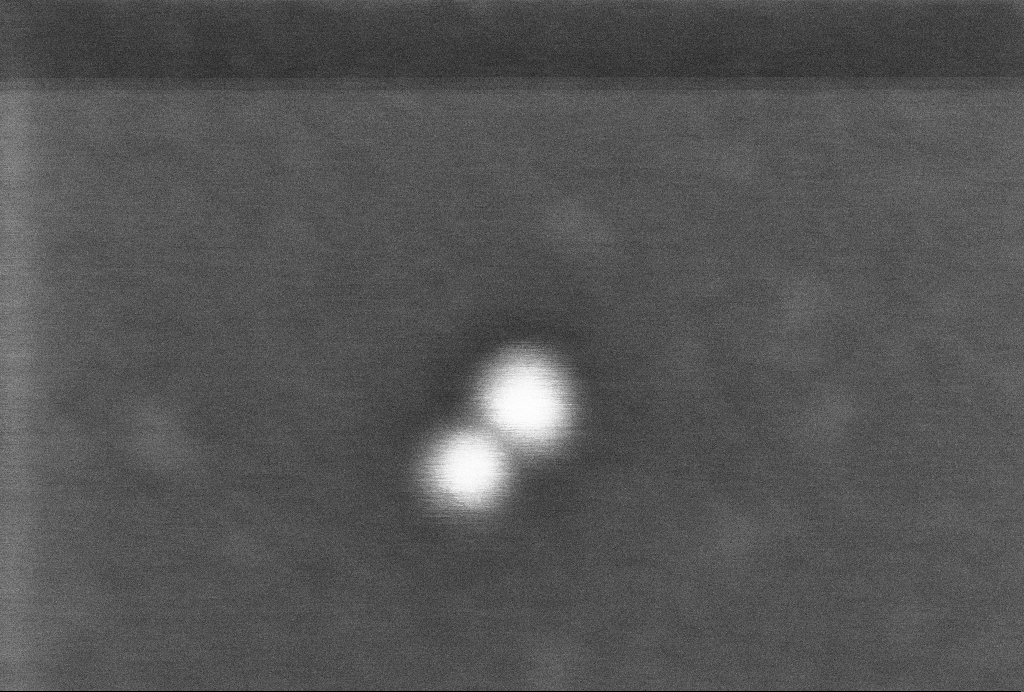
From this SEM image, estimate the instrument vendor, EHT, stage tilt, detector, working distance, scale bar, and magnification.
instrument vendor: Zeiss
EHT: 2 kV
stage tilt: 0°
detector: InLens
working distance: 3.3 mm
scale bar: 20 nm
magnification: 1030.67 K X